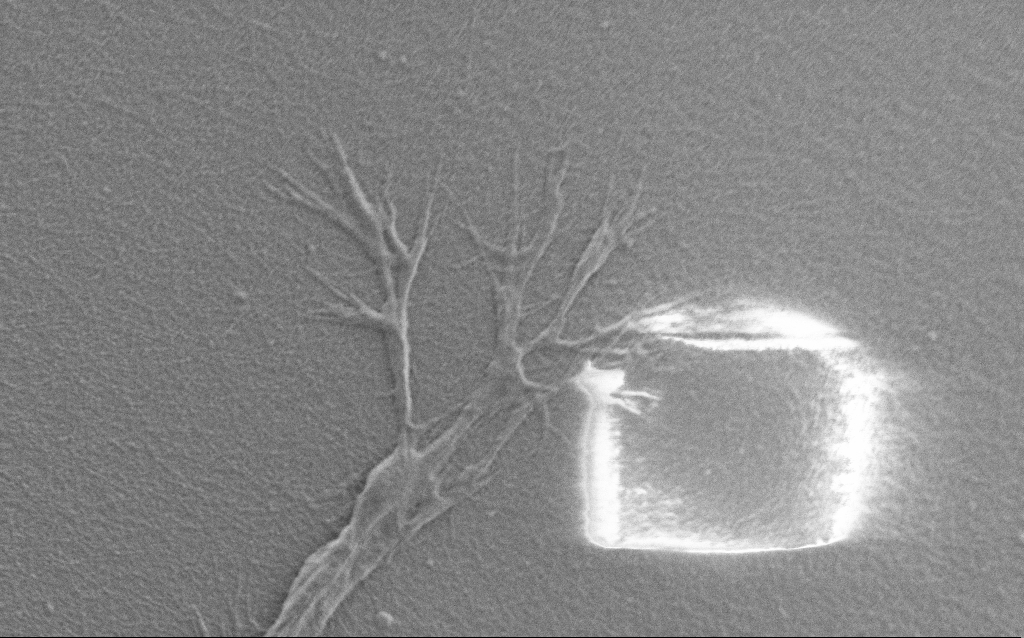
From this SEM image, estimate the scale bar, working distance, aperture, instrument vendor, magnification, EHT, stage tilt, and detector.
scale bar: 2000 nm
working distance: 7 mm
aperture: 30 µm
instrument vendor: Zeiss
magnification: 7.5 K X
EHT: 0.9 kV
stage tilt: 0°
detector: SE2